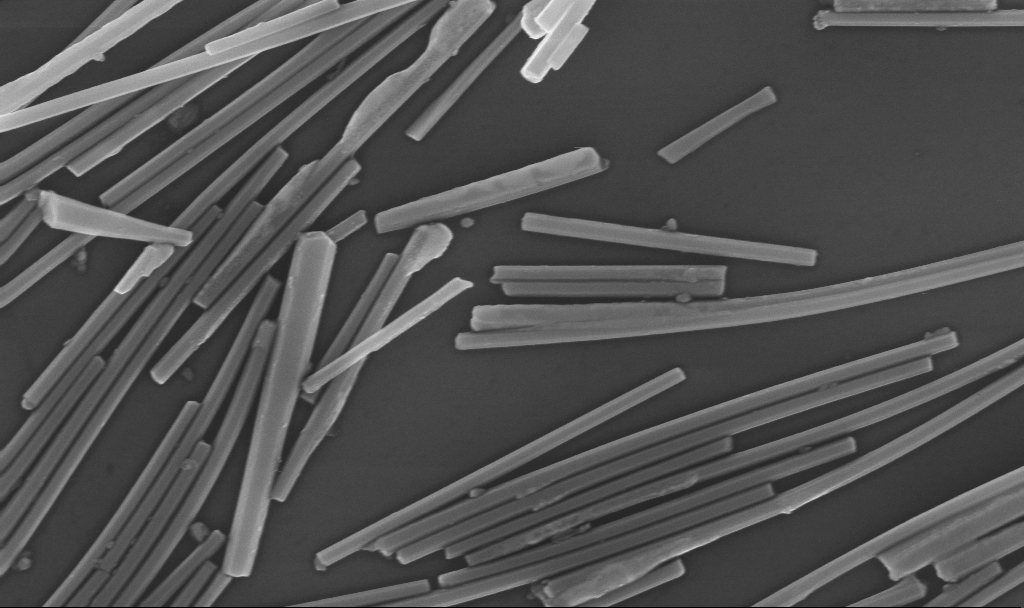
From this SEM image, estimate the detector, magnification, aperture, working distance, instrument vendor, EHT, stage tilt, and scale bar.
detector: InLens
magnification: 58.88 K X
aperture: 30 µm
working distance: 6.7 mm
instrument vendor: Zeiss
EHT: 10 kV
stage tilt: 0°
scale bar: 1000 nm